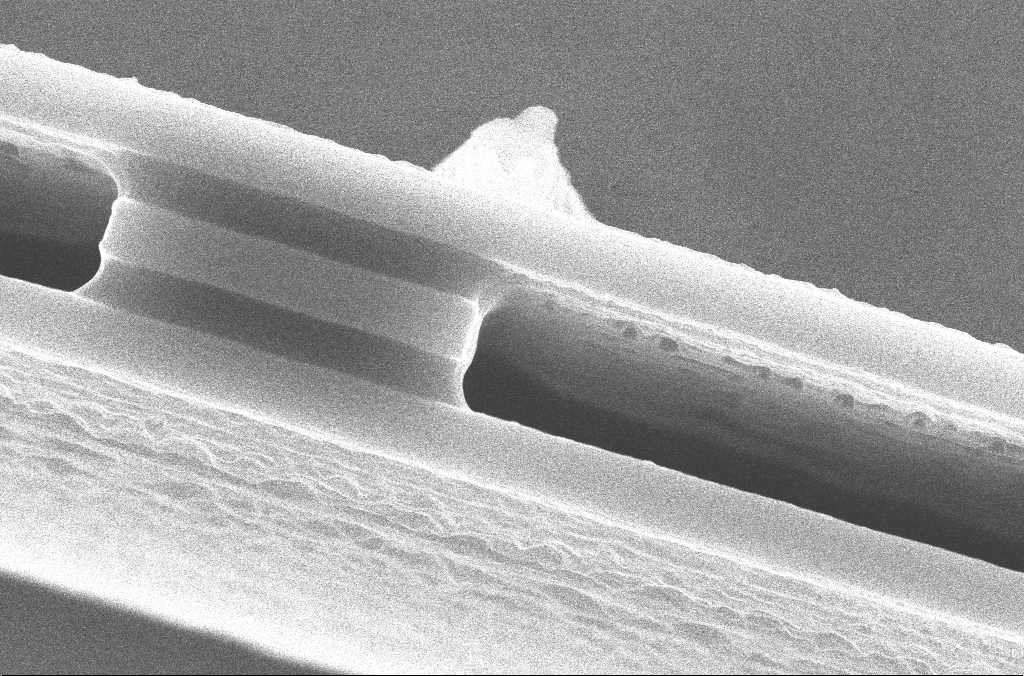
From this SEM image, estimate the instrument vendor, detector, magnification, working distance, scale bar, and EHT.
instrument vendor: Zeiss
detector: InLens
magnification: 46.08 K X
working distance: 7.1 mm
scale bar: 1000 nm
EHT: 10 kV